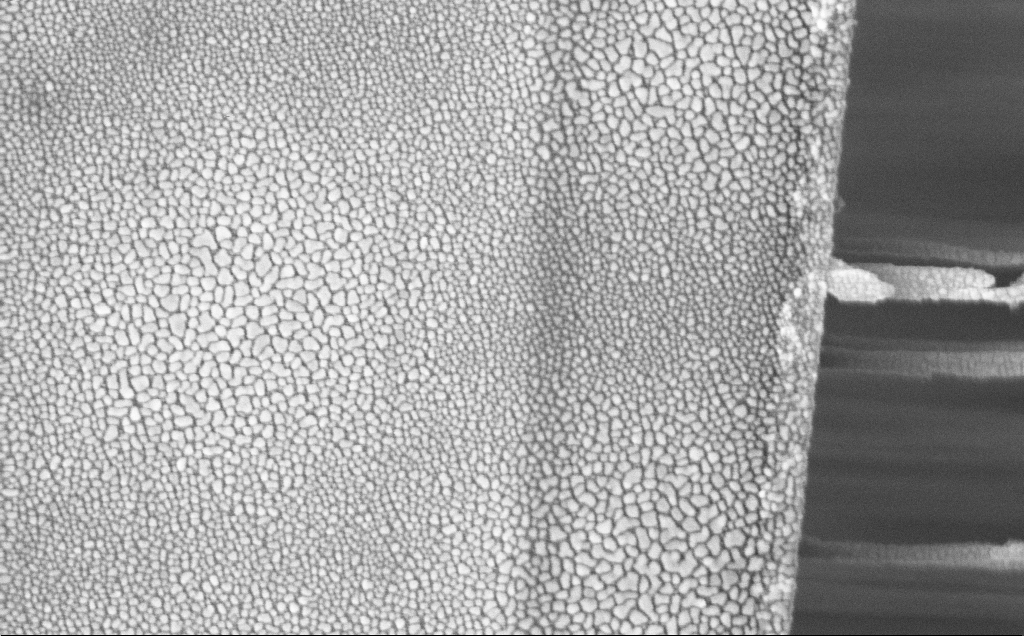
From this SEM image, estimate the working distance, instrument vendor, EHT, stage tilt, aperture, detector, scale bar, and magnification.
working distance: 12 mm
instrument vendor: Zeiss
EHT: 5 kV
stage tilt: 0°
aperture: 30 µm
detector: InLens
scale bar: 1000 nm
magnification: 62.46 K X